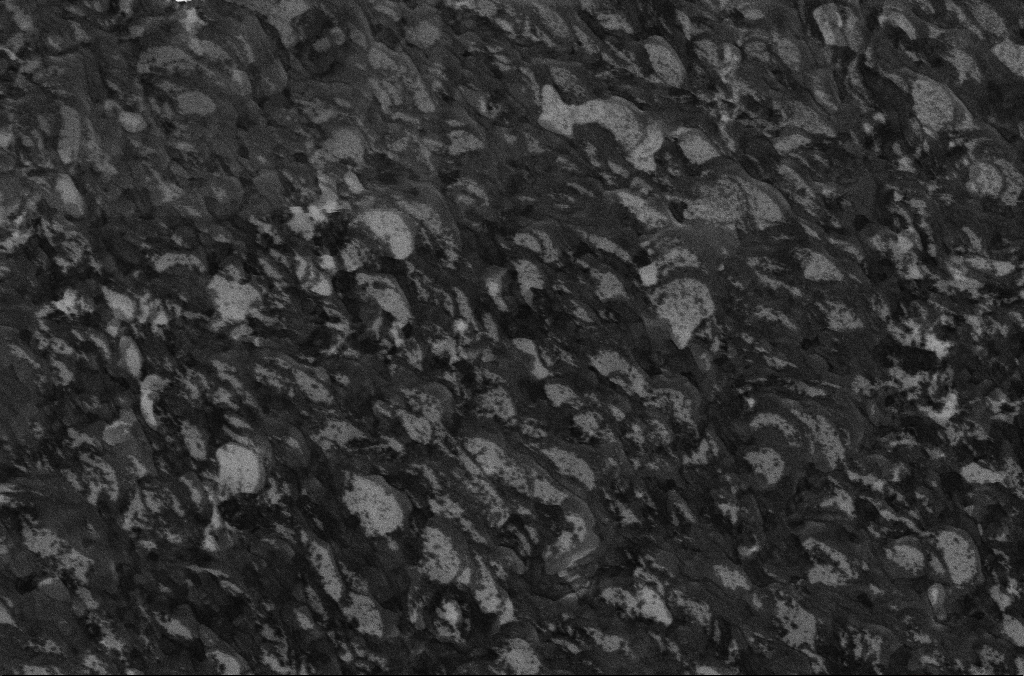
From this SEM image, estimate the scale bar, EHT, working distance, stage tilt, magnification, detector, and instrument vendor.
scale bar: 1000 nm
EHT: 5 kV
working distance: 6.7 mm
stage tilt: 0°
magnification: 20 K X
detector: InLens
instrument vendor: Zeiss